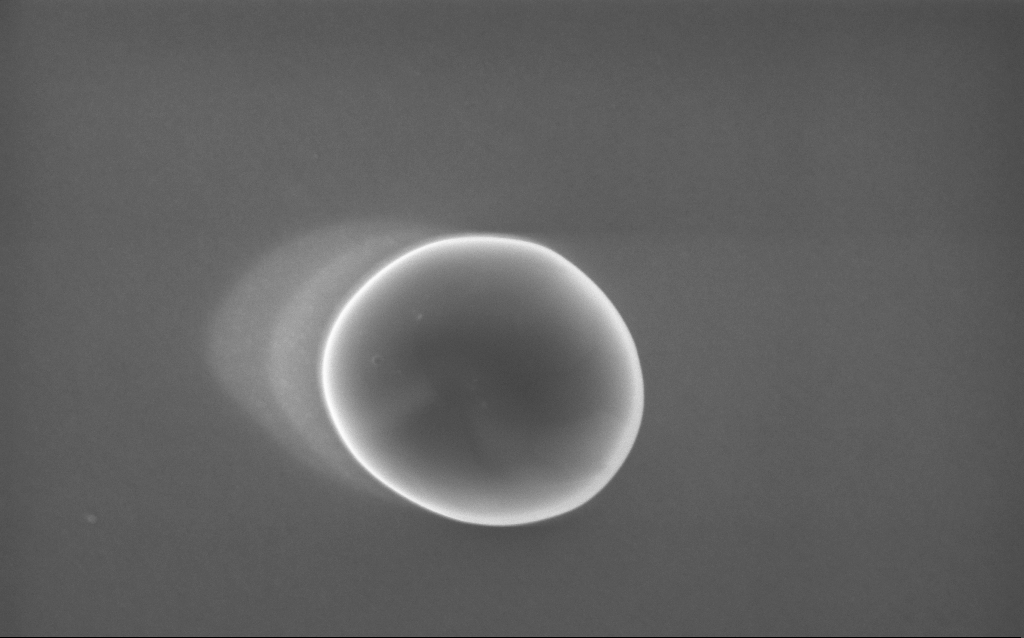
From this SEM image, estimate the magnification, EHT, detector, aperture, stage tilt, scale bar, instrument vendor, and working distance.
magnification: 106.71 K X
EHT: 10 kV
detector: InLens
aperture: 30 µm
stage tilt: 0°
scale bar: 200 nm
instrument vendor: Zeiss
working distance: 3 mm